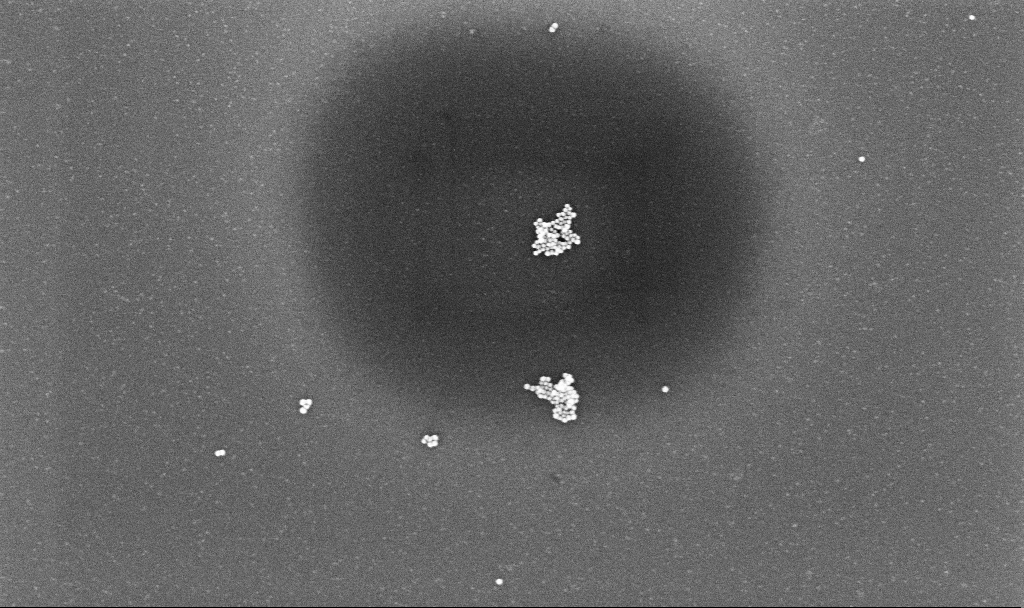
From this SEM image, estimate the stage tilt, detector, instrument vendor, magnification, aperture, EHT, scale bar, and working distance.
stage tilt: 0°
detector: InLens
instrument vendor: Zeiss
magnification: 74.62 K X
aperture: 30 µm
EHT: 10 kV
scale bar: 200 nm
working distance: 3.1 mm